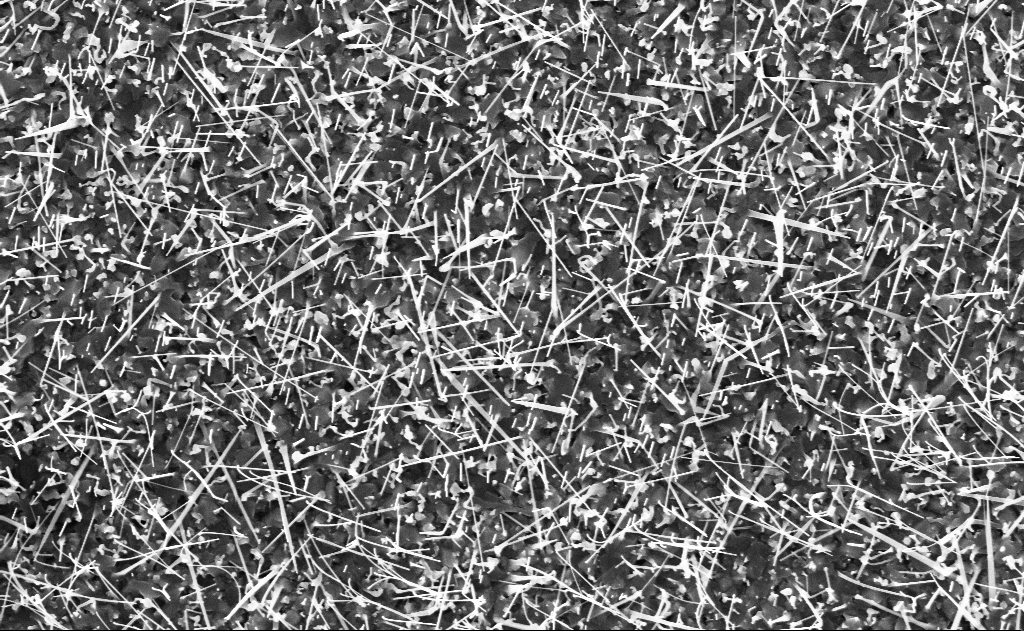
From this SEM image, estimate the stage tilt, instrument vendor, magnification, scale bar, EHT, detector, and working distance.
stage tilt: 0°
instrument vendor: Zeiss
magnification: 20 K X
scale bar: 2000 nm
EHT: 10 kV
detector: InLens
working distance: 12 mm